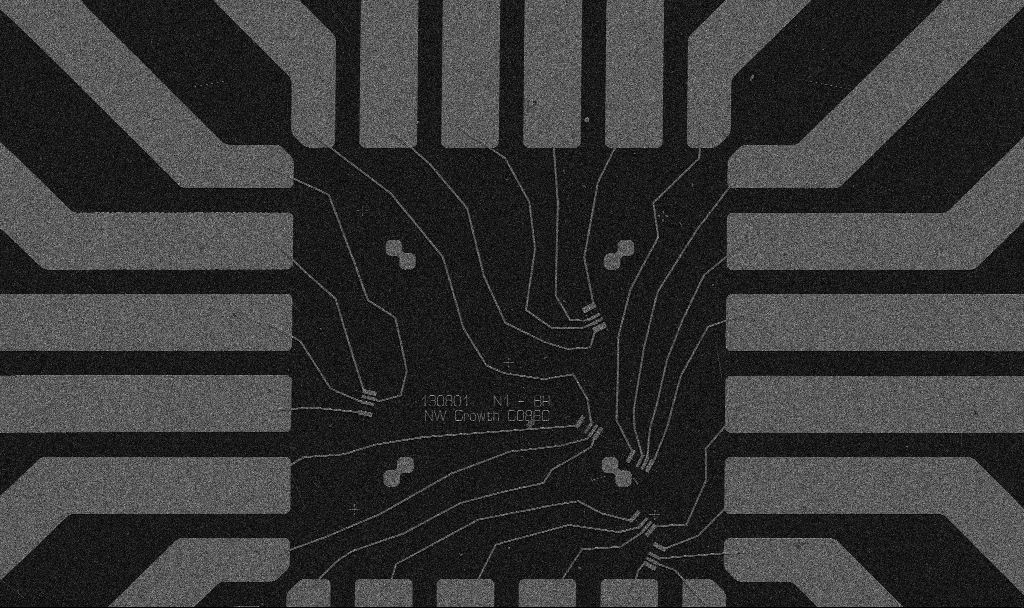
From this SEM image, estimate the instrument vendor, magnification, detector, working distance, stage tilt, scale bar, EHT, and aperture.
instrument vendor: Zeiss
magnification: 1 K X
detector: SE2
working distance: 10.7 mm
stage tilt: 0°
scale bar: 20000 nm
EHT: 5 kV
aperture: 30 µm